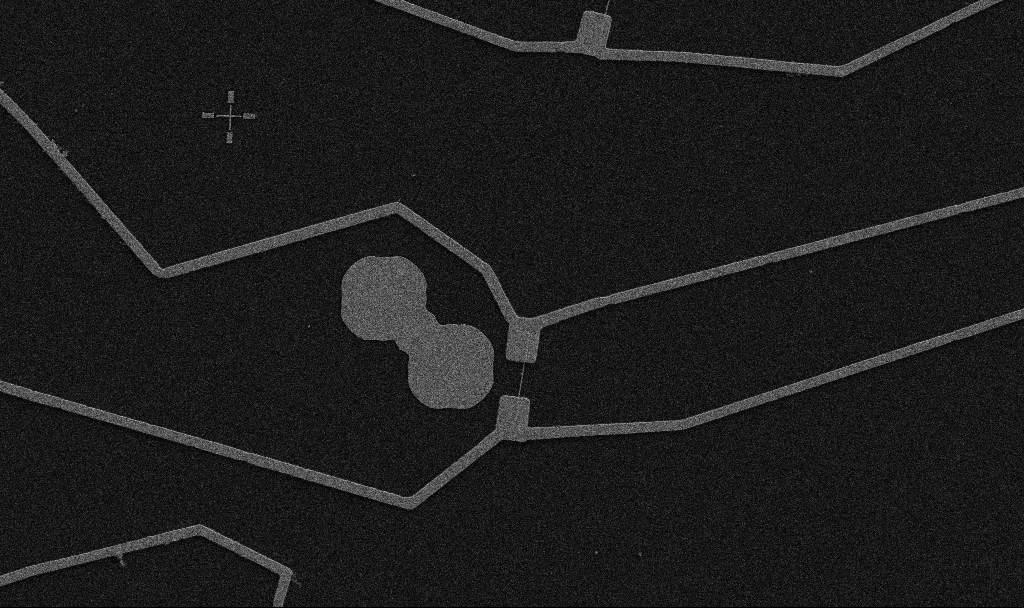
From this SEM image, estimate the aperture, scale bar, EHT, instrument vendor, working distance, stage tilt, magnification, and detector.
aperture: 30 µm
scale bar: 10000 nm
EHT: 5 kV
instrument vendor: Zeiss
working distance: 10.7 mm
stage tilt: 0°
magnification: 5 K X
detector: SE2